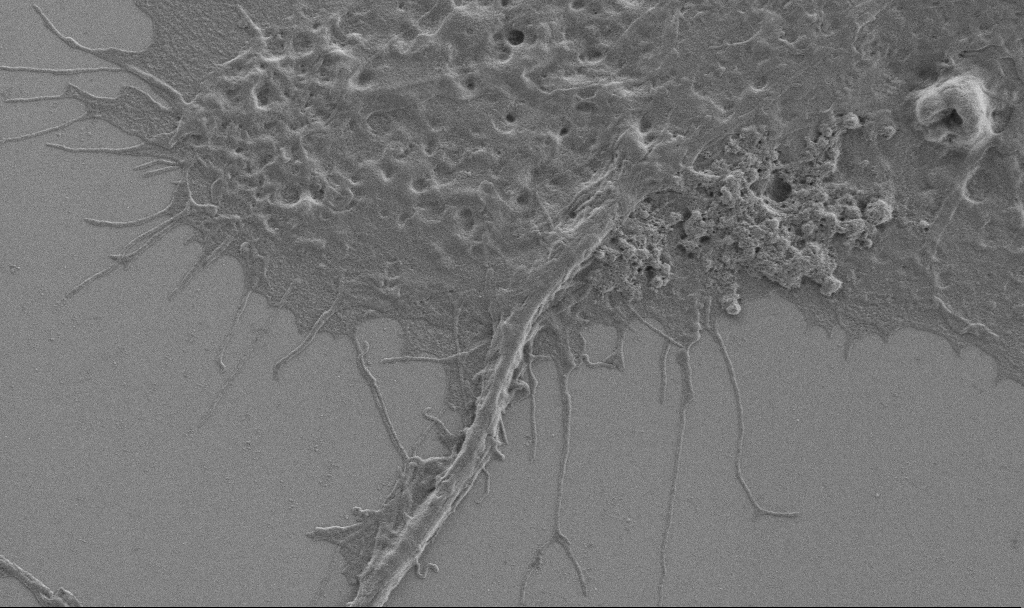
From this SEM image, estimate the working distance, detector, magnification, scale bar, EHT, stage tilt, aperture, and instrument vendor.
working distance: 6.9 mm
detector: SE2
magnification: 10 K X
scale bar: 2000 nm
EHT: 0.9 kV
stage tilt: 0°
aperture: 30 µm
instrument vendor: Zeiss